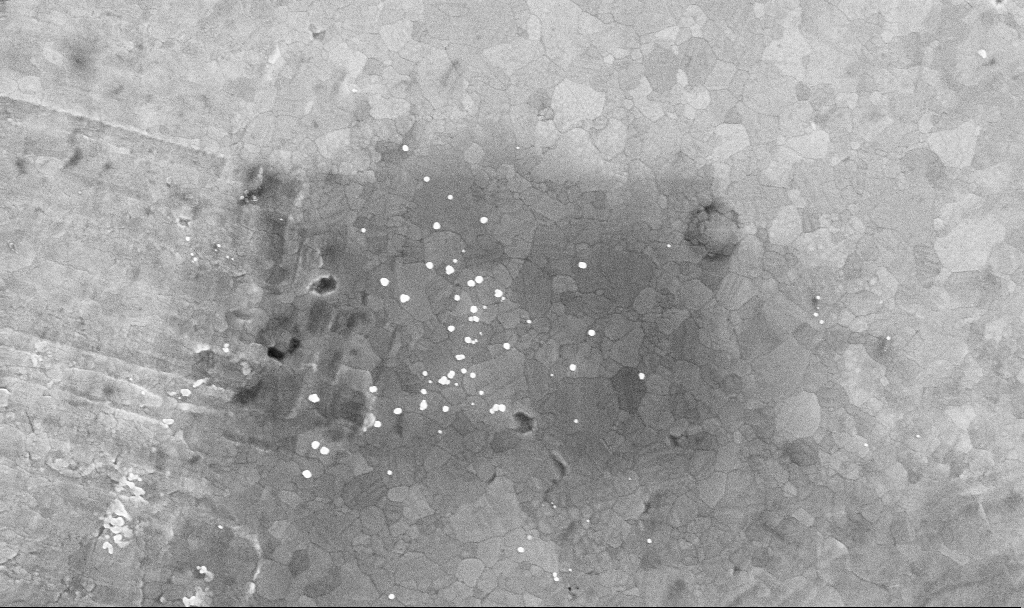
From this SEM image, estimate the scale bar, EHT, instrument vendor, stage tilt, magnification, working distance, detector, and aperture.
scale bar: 1000 nm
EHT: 10 kV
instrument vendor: Zeiss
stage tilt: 0°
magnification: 52.02 K X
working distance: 3.4 mm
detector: InLens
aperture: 30 µm